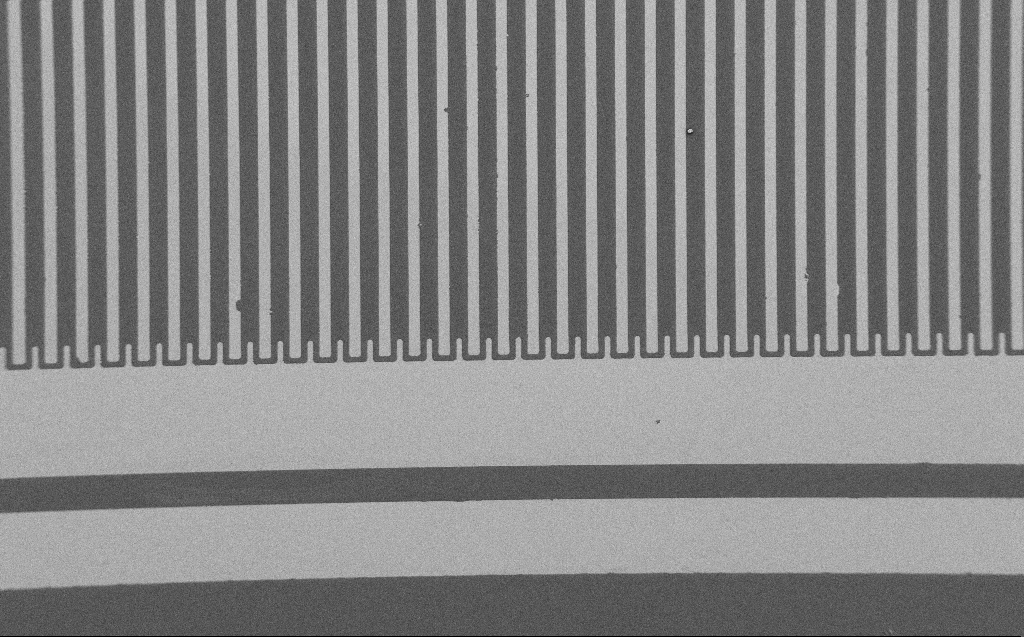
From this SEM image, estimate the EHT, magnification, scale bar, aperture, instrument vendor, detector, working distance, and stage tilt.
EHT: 1.2 kV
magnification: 0.397 K X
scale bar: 100000 nm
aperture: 30 µm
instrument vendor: Zeiss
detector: SE2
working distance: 6 mm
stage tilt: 0°